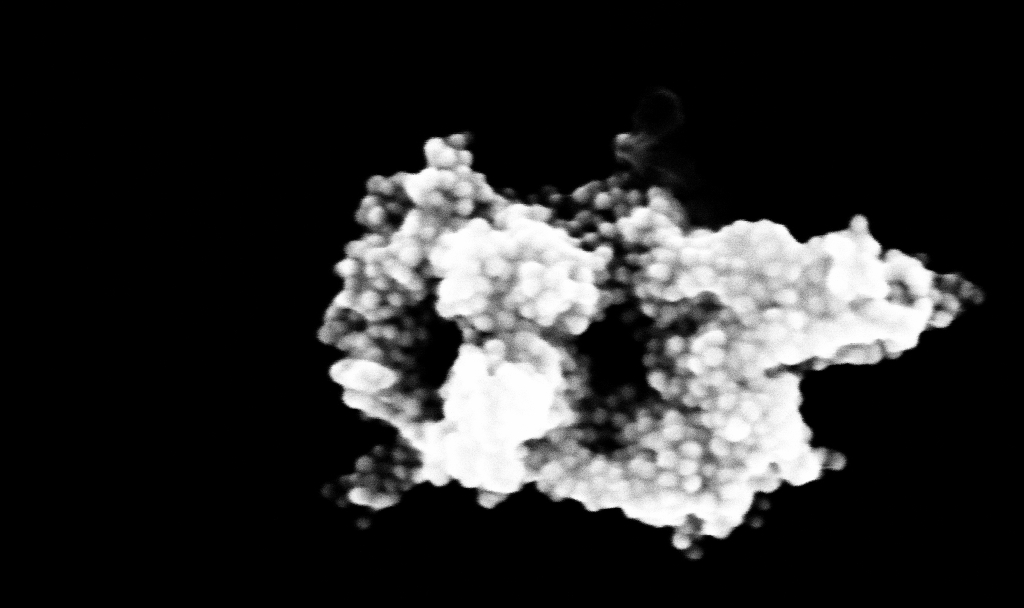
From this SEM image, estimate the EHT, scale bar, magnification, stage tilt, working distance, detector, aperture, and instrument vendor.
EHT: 10 kV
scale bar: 100 nm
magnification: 315.1 K X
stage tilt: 0°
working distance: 3.4 mm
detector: InLens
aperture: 30 µm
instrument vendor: Zeiss